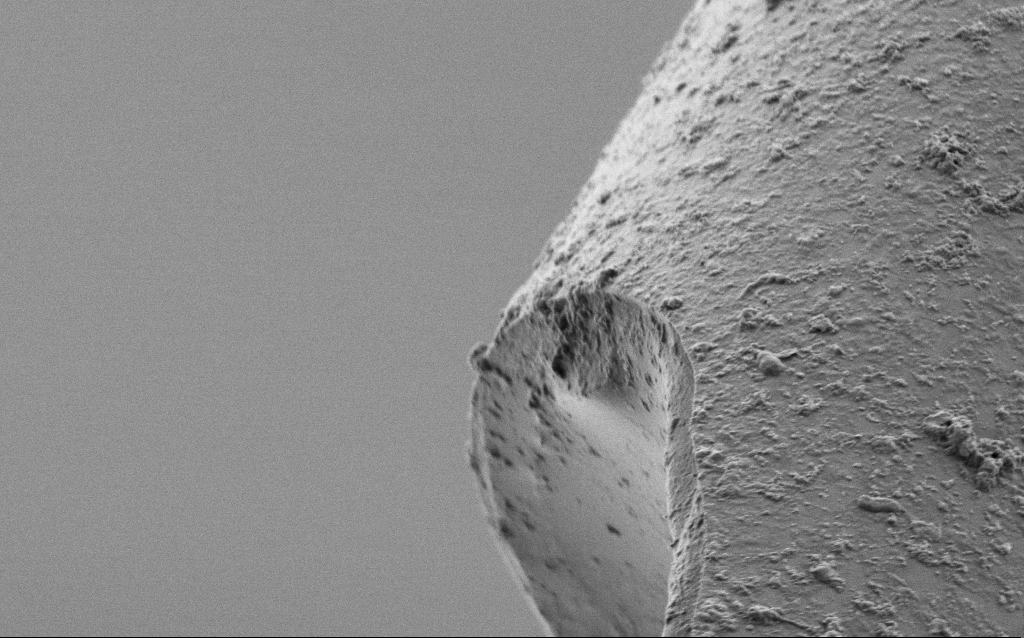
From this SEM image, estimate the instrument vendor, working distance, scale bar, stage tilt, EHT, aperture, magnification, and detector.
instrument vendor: Zeiss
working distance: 7.7 mm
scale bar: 2000 nm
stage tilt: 45°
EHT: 1 kV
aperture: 30 µm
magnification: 10 K X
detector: SE2